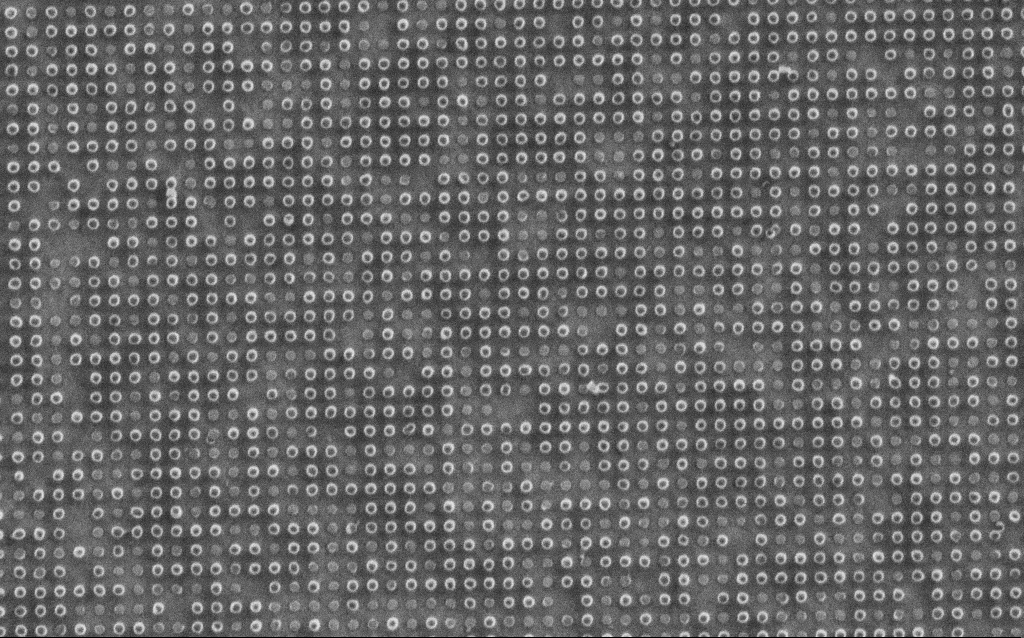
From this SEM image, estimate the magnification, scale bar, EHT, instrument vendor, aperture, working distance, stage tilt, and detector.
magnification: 50.7 K X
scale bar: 1000 nm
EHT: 1.5 kV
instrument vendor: Zeiss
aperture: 30 µm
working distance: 5.9 mm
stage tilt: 0°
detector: SE2